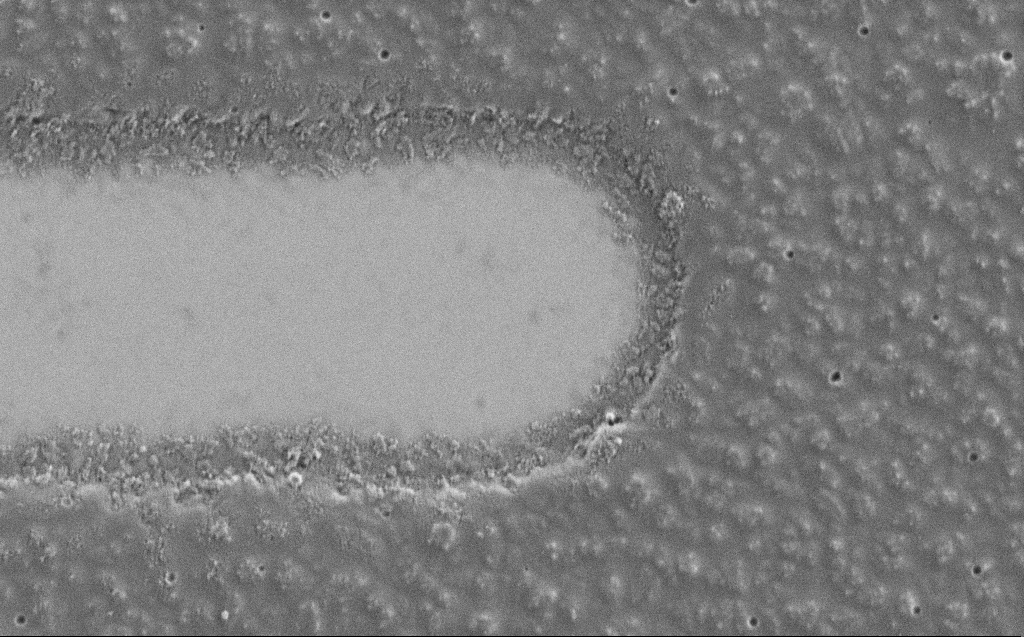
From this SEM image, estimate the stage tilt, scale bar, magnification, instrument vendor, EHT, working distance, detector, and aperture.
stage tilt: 0°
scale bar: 1000 nm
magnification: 26.79 K X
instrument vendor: Zeiss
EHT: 1.2 kV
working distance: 4 mm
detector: SE2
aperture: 30 µm